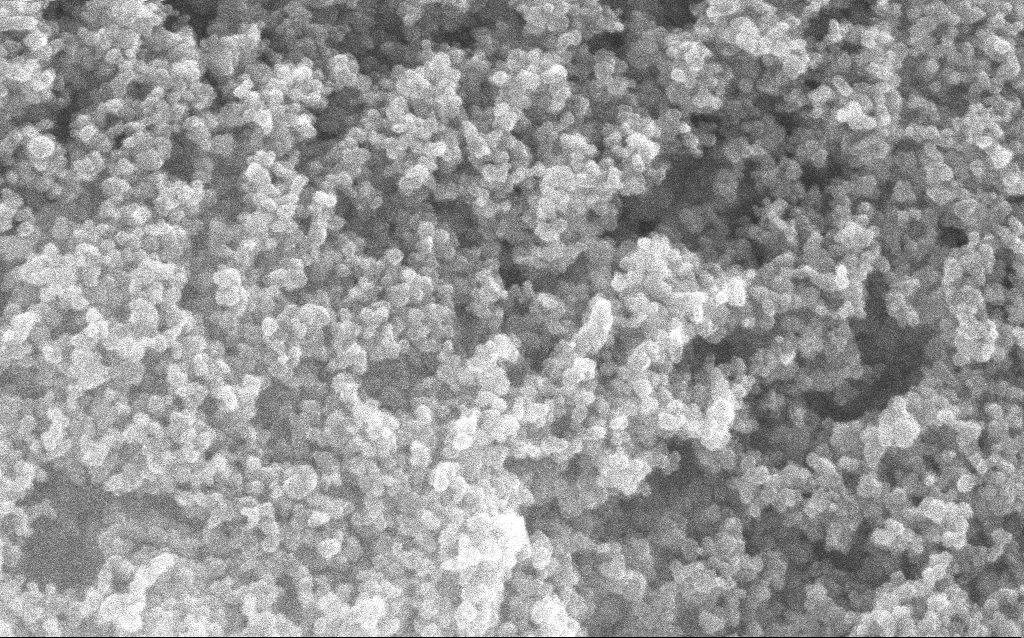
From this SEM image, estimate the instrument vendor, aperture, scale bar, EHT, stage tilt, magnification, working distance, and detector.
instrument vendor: Zeiss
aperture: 30 µm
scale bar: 100 nm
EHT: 10 kV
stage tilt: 0°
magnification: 211.33 K X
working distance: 2.4 mm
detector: InLens